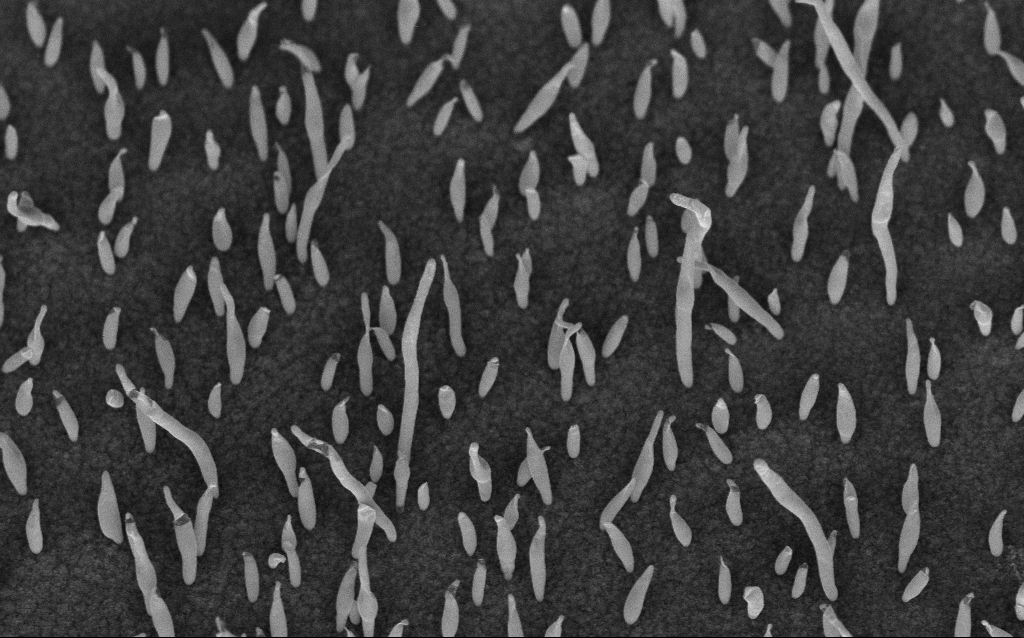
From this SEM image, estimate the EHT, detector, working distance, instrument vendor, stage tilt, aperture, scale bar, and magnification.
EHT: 5 kV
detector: InLens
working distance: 7.1 mm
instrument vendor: Zeiss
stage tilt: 45°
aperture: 30 µm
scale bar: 1000 nm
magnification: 50 K X